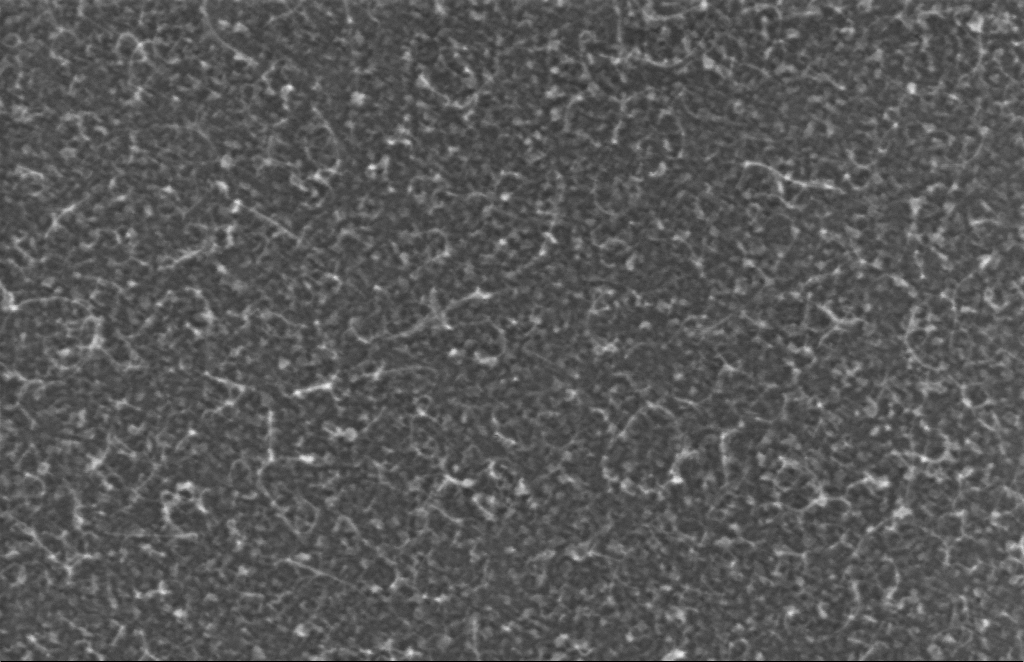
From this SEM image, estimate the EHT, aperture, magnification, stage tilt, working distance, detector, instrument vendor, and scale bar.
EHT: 5 kV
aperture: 30 µm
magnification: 334.57 K X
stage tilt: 0°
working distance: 6 mm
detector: InLens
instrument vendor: Zeiss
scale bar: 100 nm